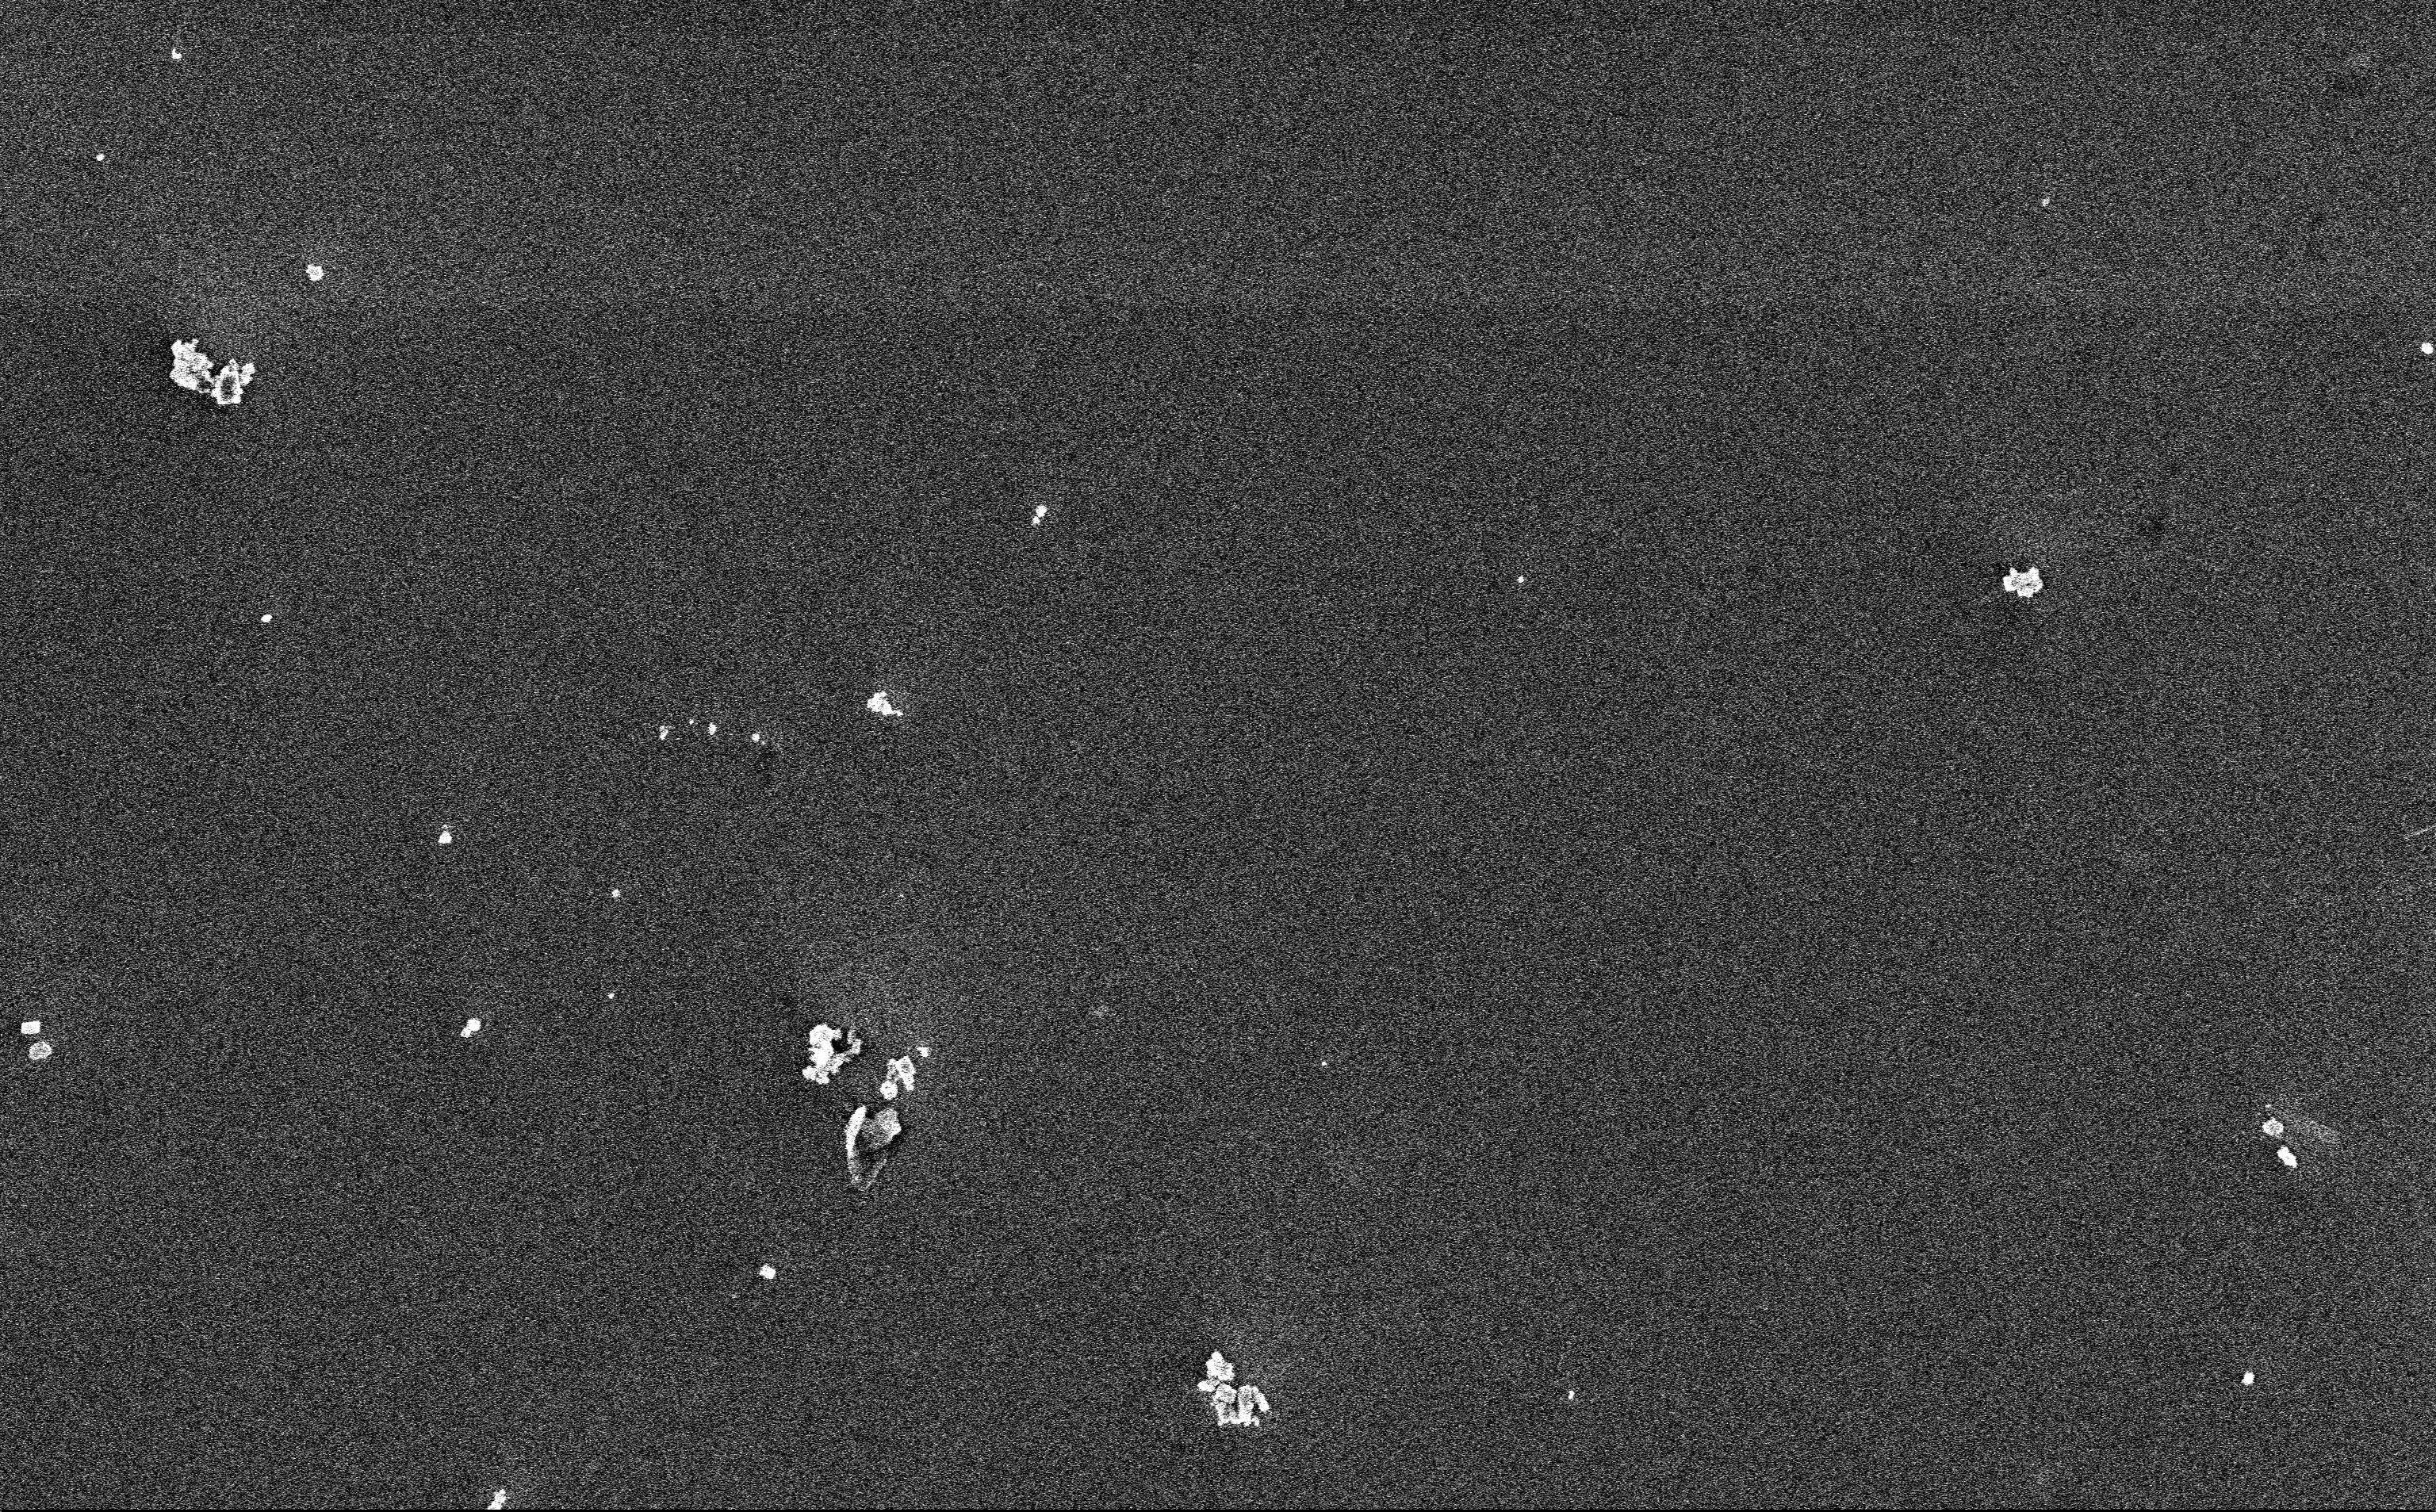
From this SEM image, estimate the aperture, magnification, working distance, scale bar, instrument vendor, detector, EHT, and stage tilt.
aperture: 30 µm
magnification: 12.85 K X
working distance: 3 mm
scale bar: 2000 nm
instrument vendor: Zeiss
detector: InLens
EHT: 3 kV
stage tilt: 0°